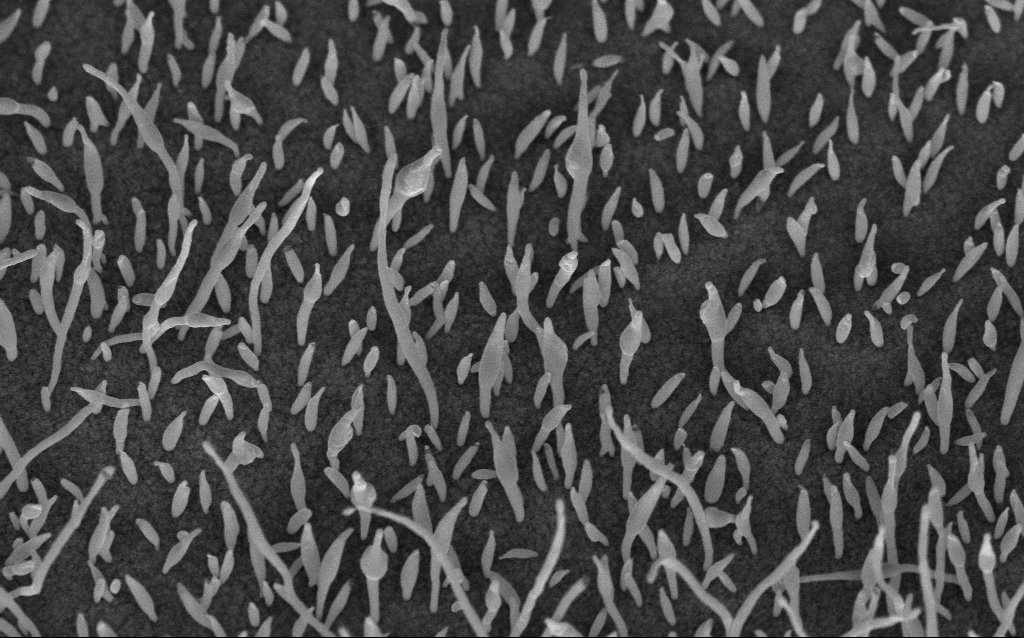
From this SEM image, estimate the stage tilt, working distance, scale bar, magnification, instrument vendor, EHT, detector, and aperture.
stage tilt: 45°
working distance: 6.4 mm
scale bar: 1000 nm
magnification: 50 K X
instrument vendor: Zeiss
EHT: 5 kV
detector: InLens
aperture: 30 µm